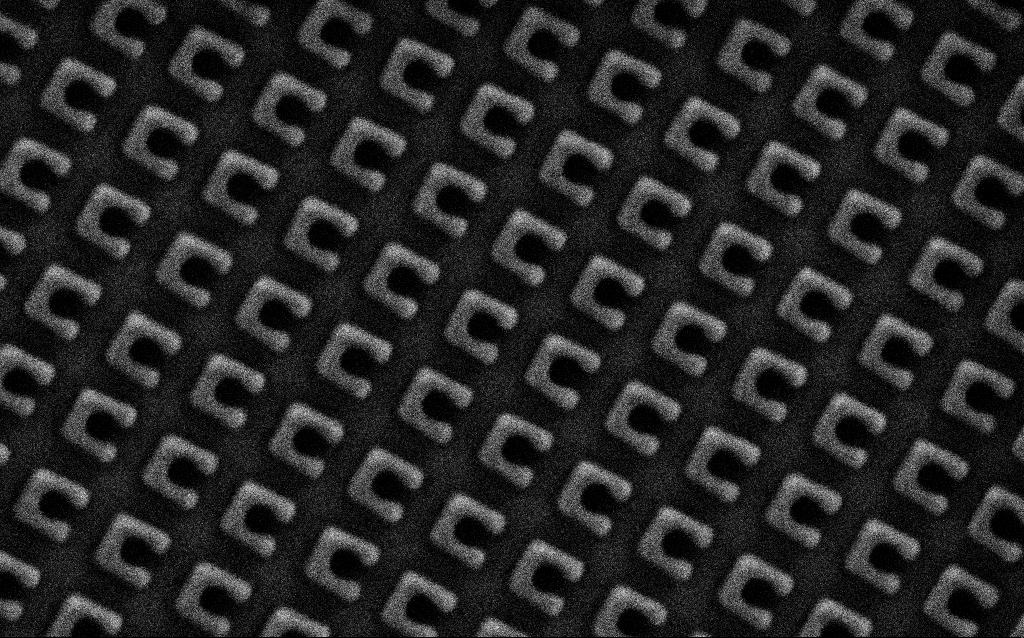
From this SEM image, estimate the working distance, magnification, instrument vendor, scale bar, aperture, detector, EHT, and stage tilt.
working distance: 8 mm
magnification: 73.22 K X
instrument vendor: Zeiss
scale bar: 200 nm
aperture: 30 µm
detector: SE2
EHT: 1.5 kV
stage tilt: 0°